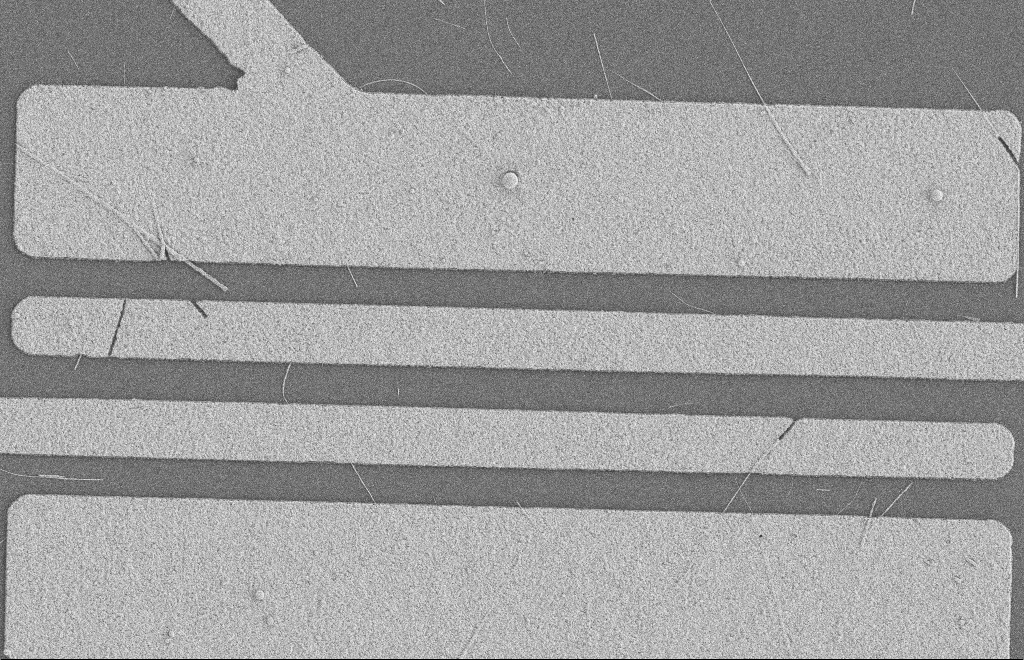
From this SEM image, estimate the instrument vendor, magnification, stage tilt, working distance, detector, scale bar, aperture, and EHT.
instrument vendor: Zeiss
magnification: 6.04 K X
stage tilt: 0°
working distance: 8 mm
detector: SE2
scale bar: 2000 nm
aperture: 20 µm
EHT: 2 kV